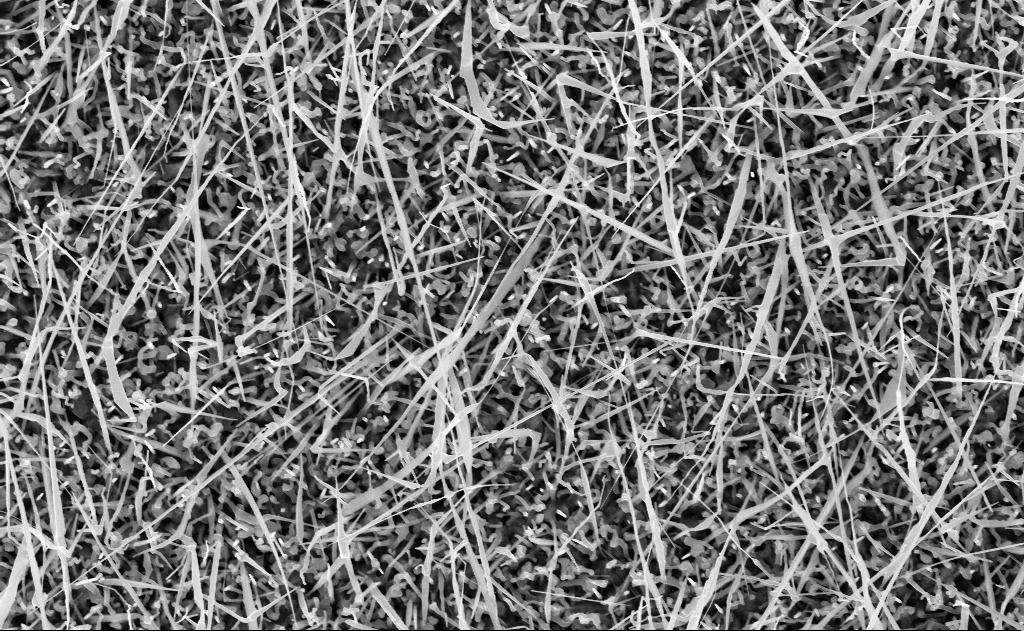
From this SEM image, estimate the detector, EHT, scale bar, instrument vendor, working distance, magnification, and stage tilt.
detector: InLens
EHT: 10 kV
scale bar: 2000 nm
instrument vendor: Zeiss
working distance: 10 mm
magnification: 20 K X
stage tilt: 0°